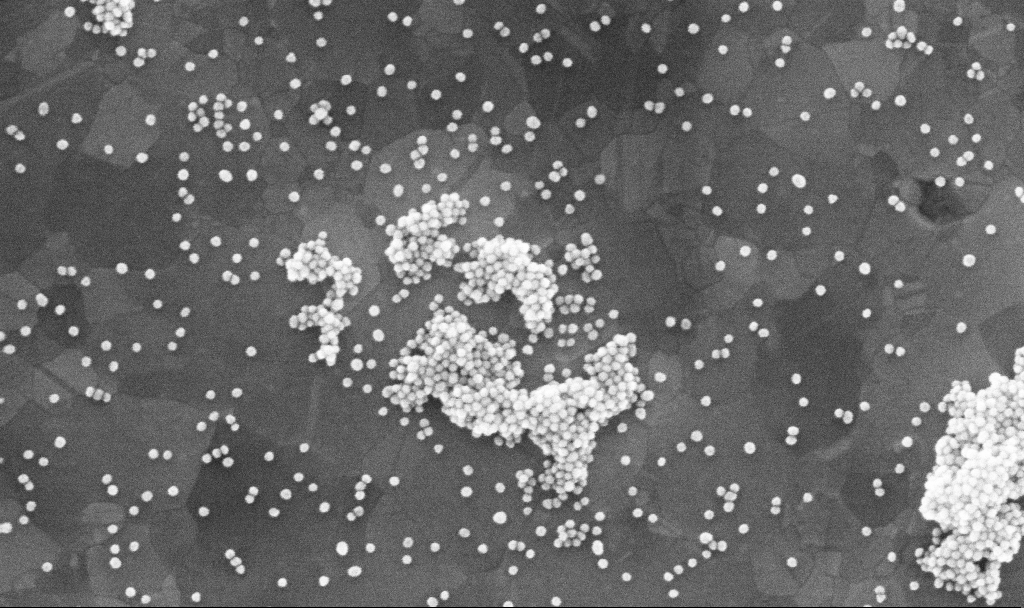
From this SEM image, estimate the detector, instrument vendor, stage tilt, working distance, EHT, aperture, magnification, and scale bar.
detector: InLens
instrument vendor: Zeiss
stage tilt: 0°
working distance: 3.3 mm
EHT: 10 kV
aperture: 30 µm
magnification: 200 K X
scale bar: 100 nm